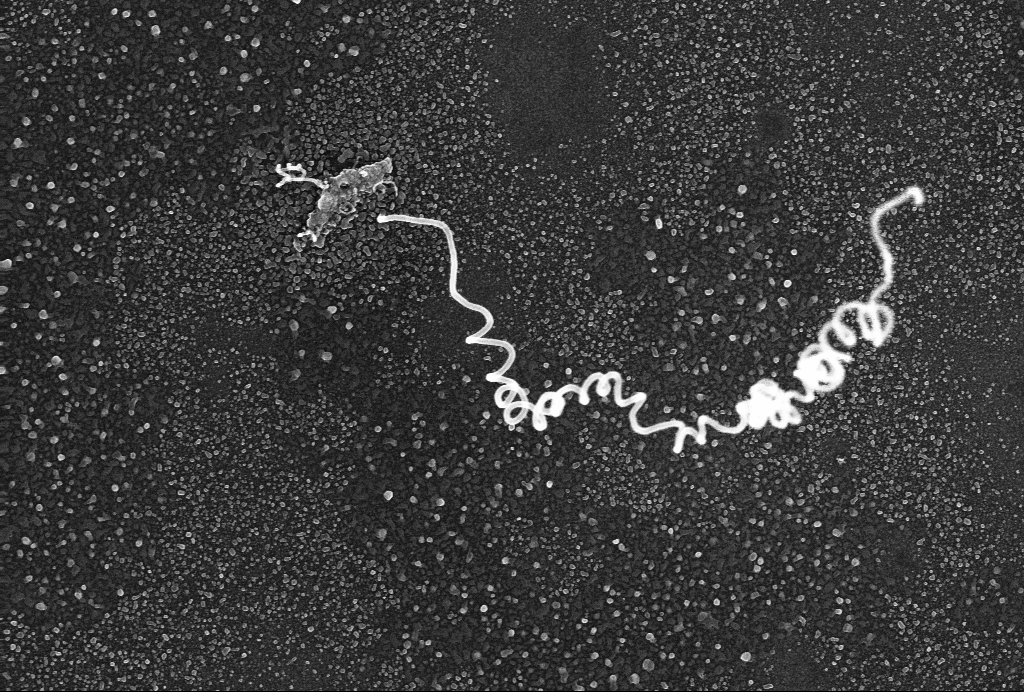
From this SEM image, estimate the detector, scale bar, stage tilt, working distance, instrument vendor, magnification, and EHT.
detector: InLens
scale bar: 300 nm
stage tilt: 0°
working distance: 3 mm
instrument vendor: Zeiss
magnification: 57.88 K X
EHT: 10 kV